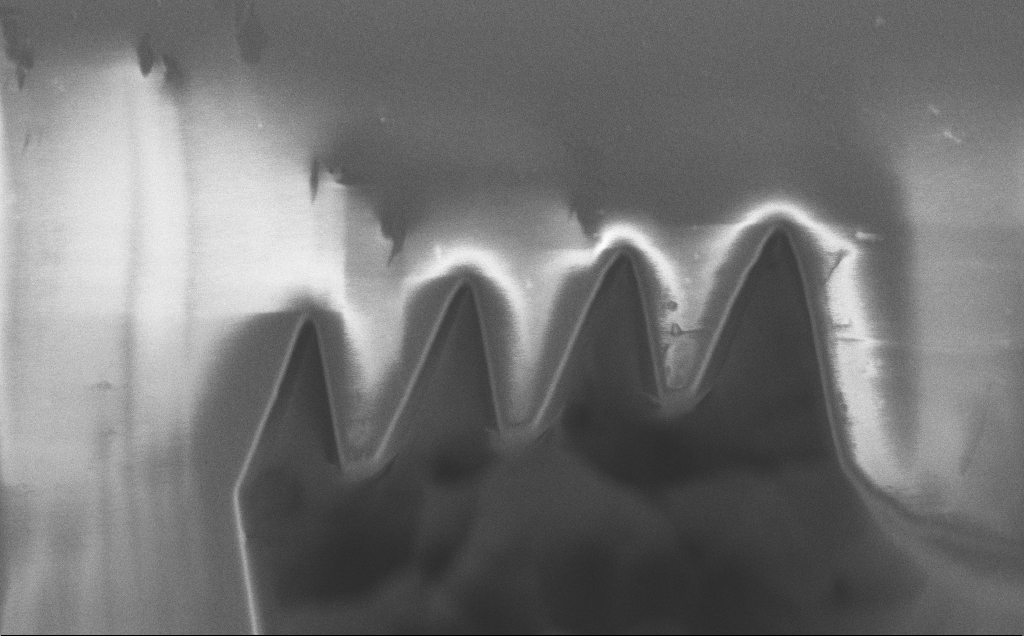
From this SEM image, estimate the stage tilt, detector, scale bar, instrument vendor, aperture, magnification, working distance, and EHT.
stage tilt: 0°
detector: SE2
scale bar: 10000 nm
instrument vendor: Zeiss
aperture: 30 µm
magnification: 5.1 K X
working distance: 6 mm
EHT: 1.2 kV